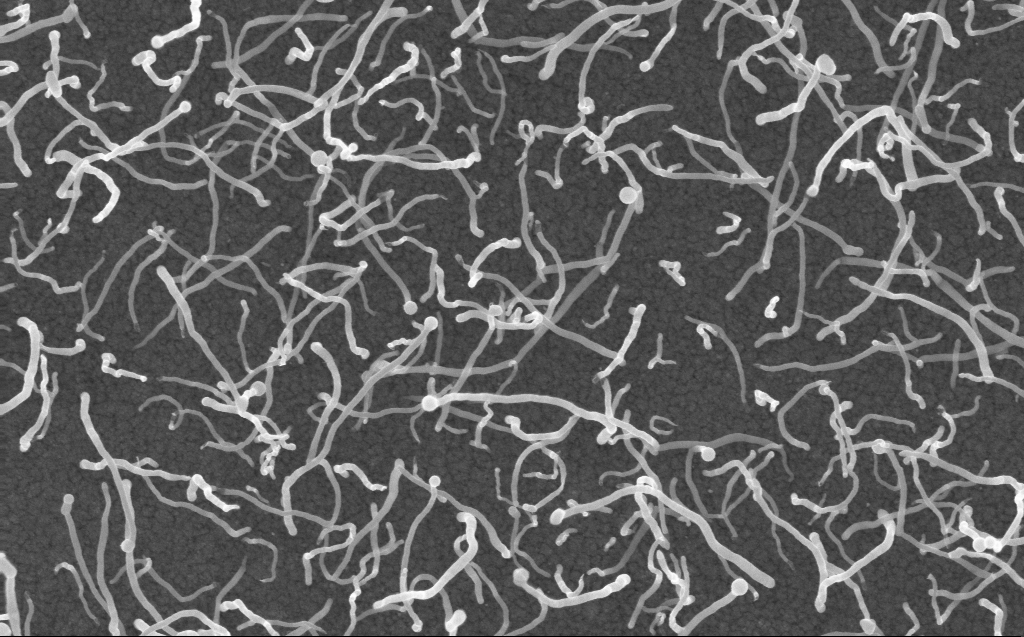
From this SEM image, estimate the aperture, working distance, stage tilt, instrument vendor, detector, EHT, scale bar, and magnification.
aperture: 30 µm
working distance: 3 mm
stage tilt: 0°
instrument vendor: Zeiss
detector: InLens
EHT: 10 kV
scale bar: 1000 nm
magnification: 50 K X